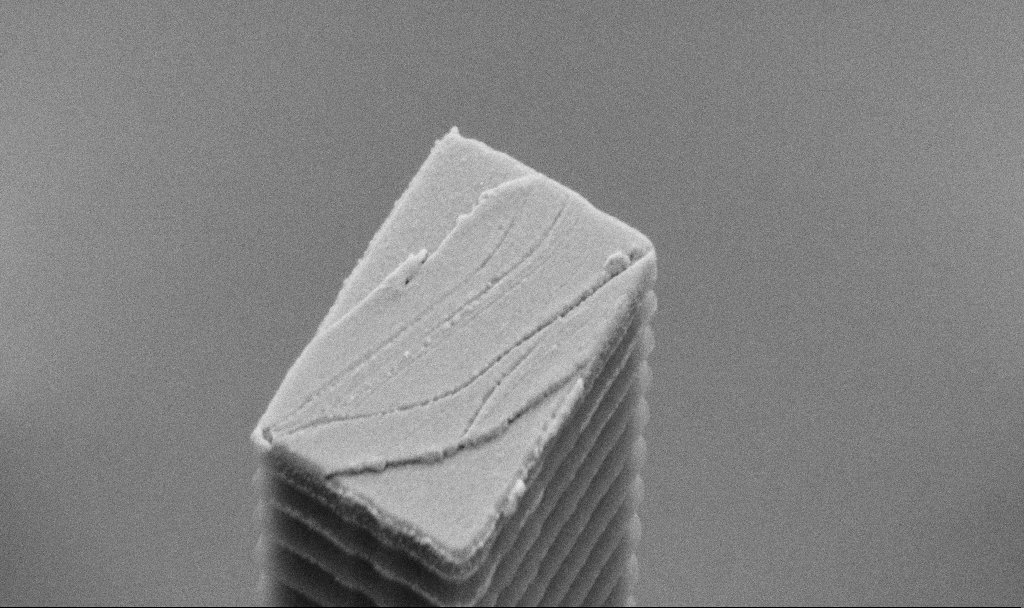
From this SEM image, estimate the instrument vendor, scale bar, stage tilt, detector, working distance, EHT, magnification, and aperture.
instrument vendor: Zeiss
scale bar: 1000 nm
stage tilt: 44.9°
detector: SE2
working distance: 5.7 mm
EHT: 5 kV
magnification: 48.44 K X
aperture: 30 µm